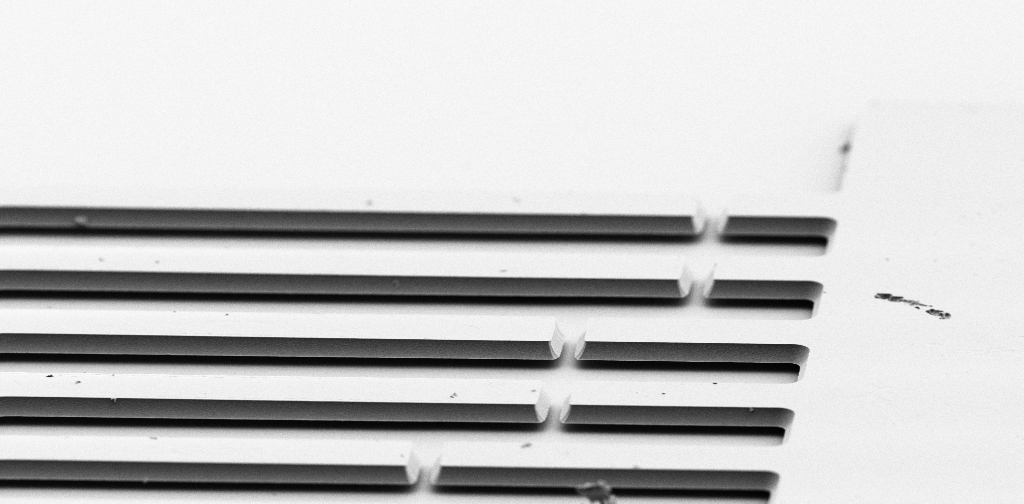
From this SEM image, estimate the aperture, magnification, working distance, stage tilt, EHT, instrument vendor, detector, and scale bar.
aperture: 30 µm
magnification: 1.7 K X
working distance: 5 mm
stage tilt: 70°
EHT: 3 kV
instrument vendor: Zeiss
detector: SE2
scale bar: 10000 nm